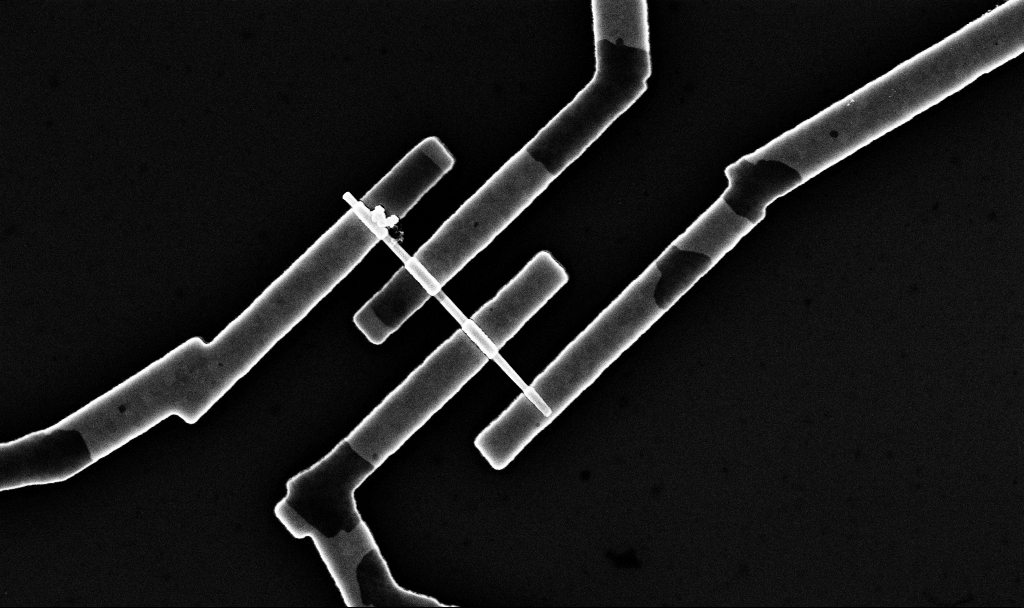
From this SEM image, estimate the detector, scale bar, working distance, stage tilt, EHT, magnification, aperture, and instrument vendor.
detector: InLens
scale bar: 2000 nm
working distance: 6.7 mm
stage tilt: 0°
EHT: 10 kV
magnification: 27.19 K X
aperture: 30 µm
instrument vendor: Zeiss